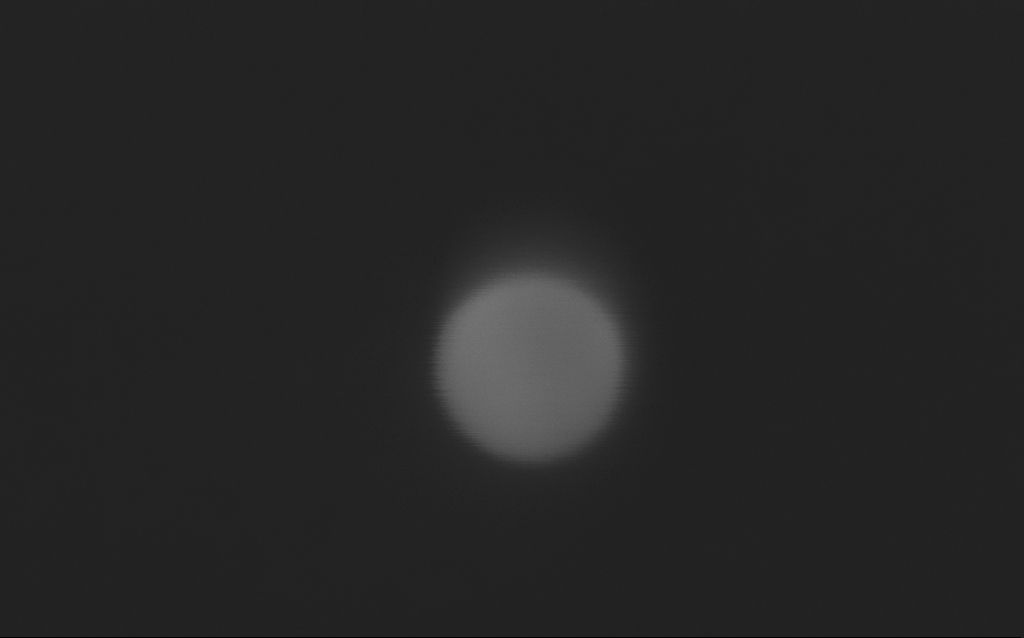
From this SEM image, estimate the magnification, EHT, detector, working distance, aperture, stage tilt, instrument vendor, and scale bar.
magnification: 1103.86 K X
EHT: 5 kV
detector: InLens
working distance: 3 mm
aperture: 30 µm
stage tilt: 0°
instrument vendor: Zeiss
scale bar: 20 nm